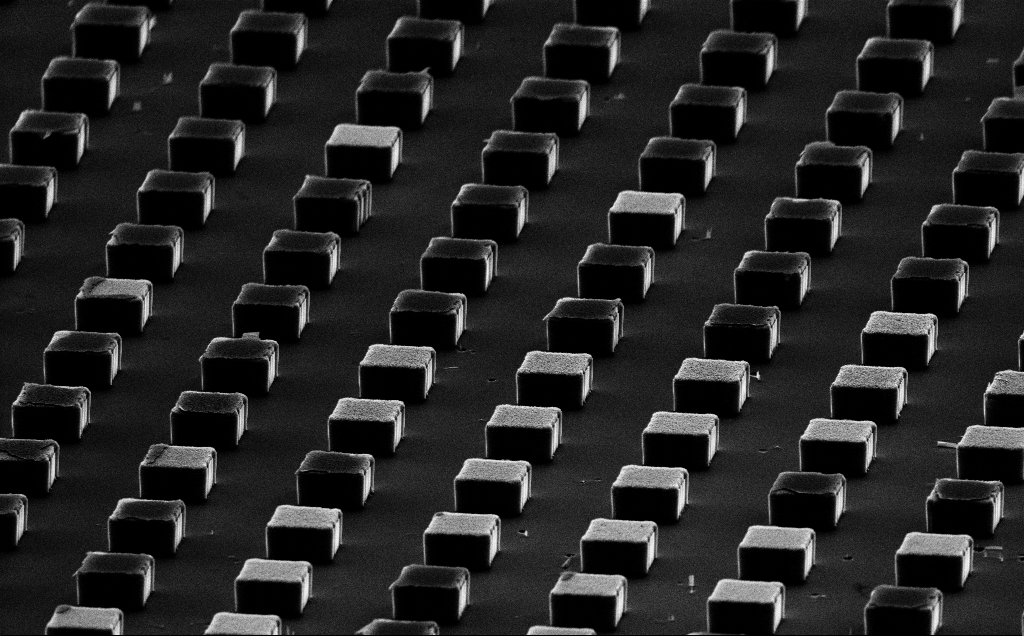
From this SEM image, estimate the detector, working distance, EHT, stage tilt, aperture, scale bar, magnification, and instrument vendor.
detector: SE2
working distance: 18 mm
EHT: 10 kV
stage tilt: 70°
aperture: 30 µm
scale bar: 10000 nm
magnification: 2.93 K X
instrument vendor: Zeiss